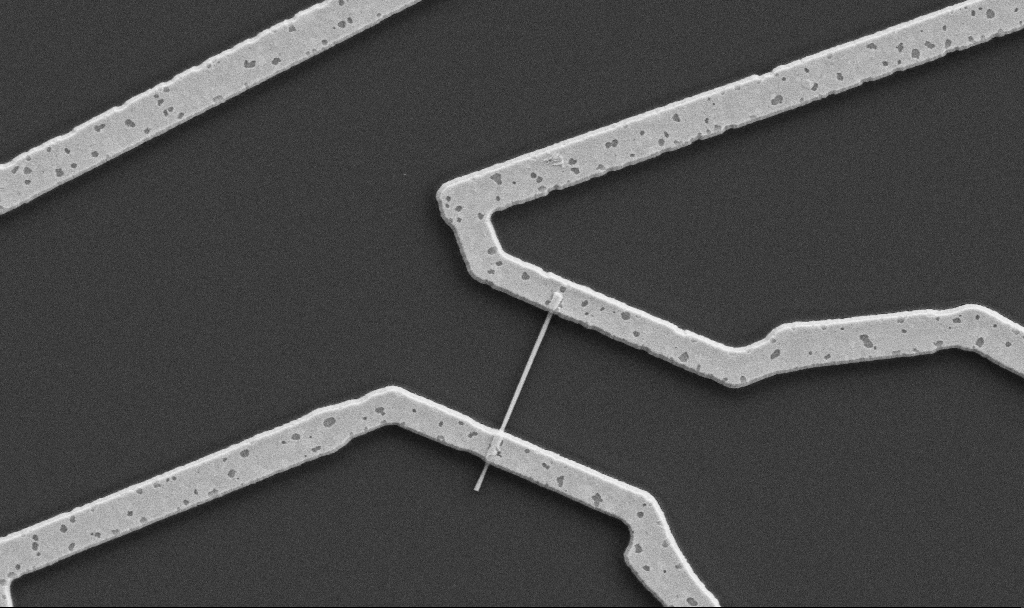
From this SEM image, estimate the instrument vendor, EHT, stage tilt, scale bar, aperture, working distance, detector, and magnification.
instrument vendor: Zeiss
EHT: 5 kV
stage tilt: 0°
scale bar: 1000 nm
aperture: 30 µm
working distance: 10.7 mm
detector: SE2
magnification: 20 K X